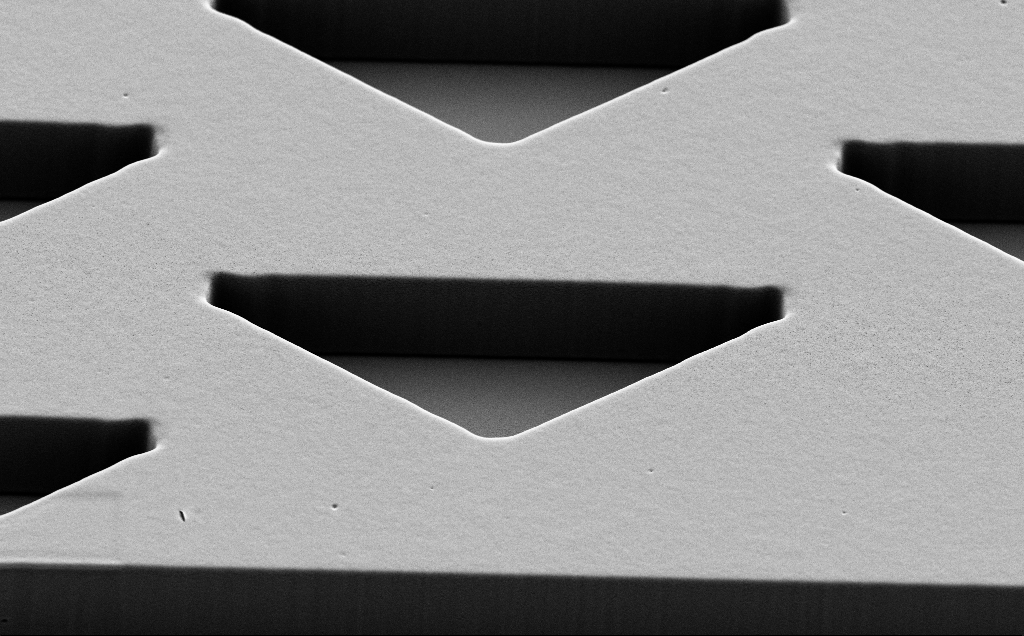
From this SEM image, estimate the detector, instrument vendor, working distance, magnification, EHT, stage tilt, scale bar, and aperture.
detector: SE2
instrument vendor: Zeiss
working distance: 9 mm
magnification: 5.93 K X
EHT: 5 kV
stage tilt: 40°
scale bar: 10000 nm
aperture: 30 µm